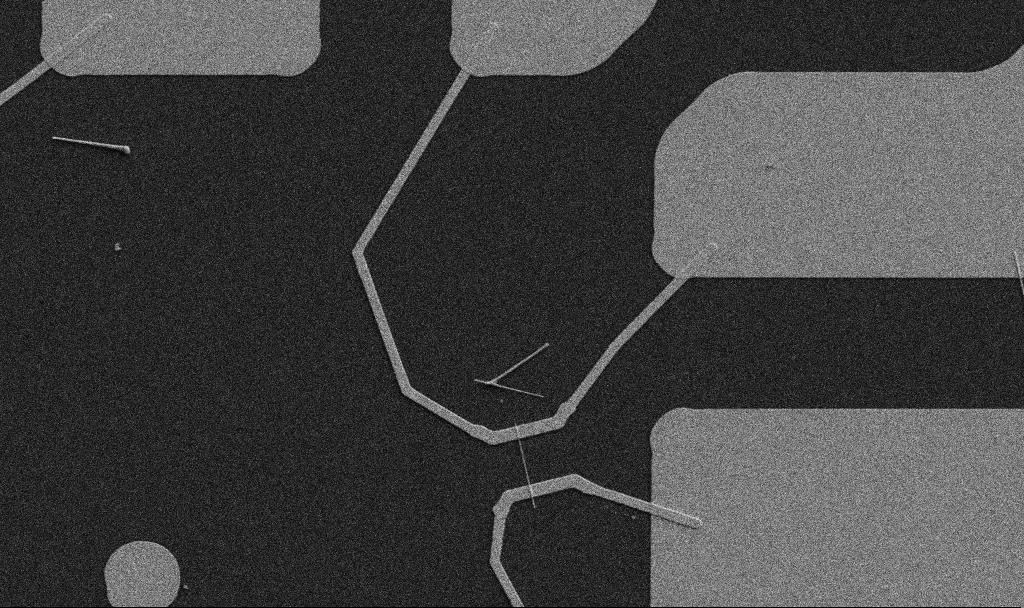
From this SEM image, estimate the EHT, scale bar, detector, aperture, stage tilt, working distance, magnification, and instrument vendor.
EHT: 5 kV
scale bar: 10000 nm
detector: SE2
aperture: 30 µm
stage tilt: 0°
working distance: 10.7 mm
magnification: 5 K X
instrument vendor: Zeiss